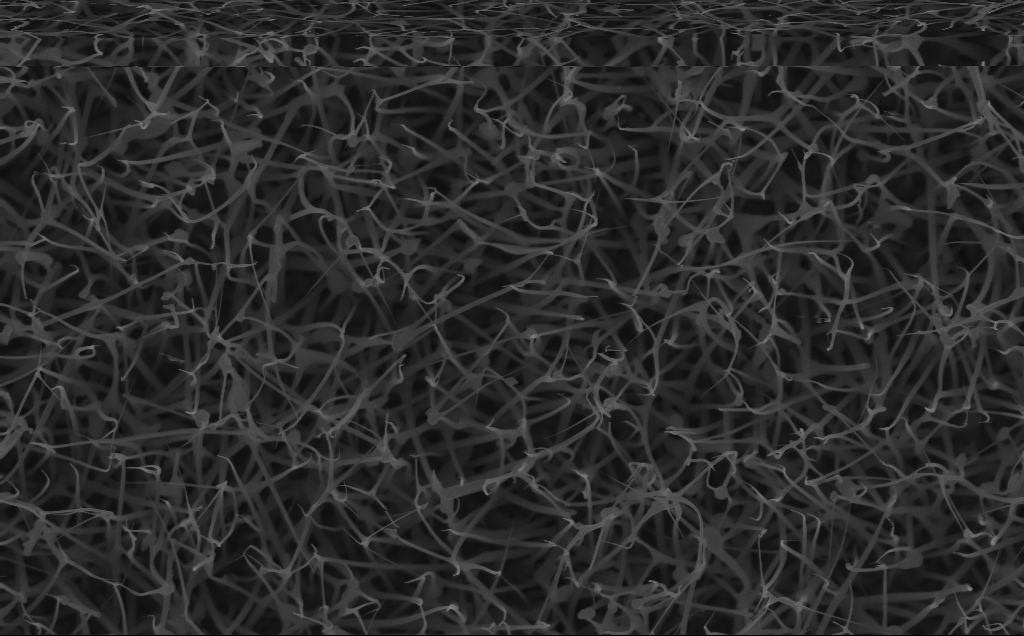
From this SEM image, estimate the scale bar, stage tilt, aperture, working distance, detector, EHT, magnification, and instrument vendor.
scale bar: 1000 nm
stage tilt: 0°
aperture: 30 µm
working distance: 6 mm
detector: InLens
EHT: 10 kV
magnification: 20 K X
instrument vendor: Zeiss